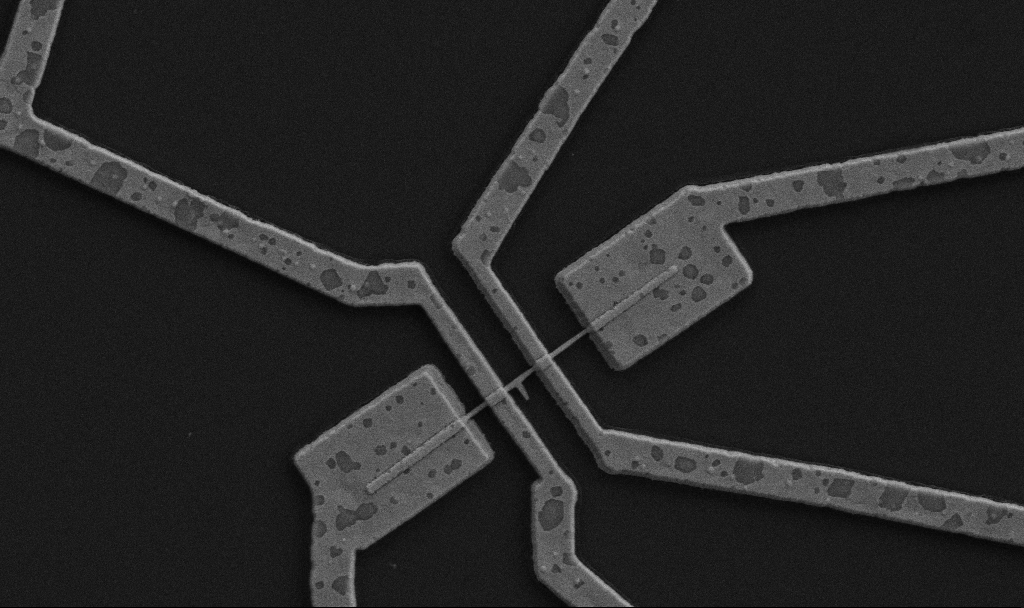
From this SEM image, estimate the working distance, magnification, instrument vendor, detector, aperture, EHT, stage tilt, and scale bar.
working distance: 10.7 mm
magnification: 20 K X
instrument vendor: Zeiss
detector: SE2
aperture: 30 µm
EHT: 5 kV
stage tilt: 0°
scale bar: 1000 nm